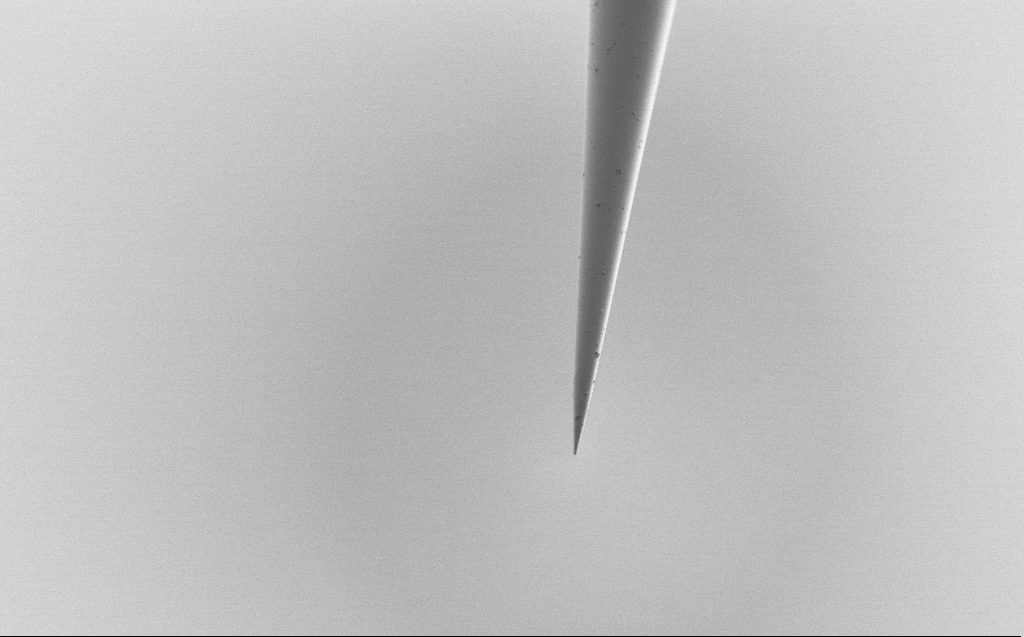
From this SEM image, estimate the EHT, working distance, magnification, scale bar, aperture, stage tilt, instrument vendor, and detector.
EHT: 1 kV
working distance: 5 mm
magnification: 5 K X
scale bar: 10000 nm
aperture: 30 µm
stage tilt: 45°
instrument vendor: Zeiss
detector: SE2